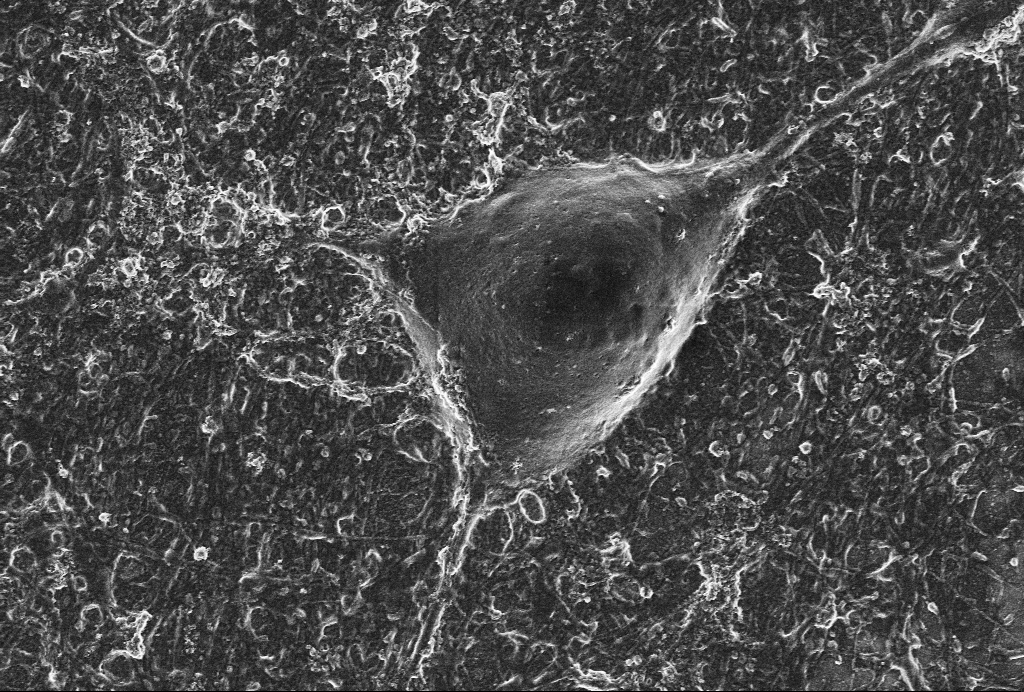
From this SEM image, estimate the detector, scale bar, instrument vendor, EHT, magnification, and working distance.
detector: InLens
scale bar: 2000 nm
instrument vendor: Zeiss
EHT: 2 kV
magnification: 10 K X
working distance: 6 mm